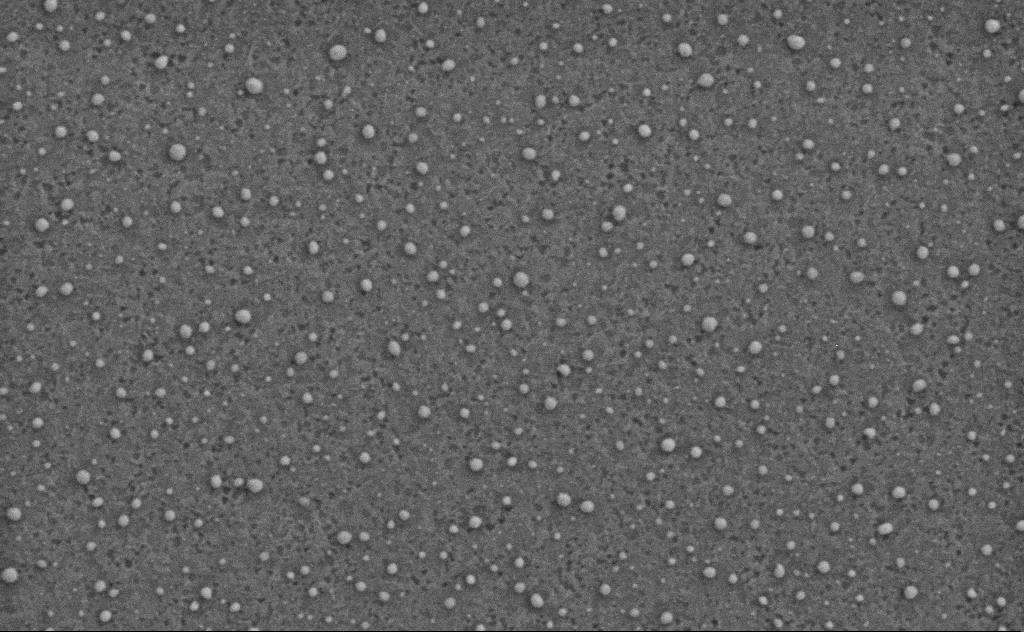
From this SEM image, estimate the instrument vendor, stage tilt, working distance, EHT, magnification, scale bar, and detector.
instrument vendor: Zeiss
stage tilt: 0°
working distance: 4 mm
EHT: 3 kV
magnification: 80 K X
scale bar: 200 nm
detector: SE2